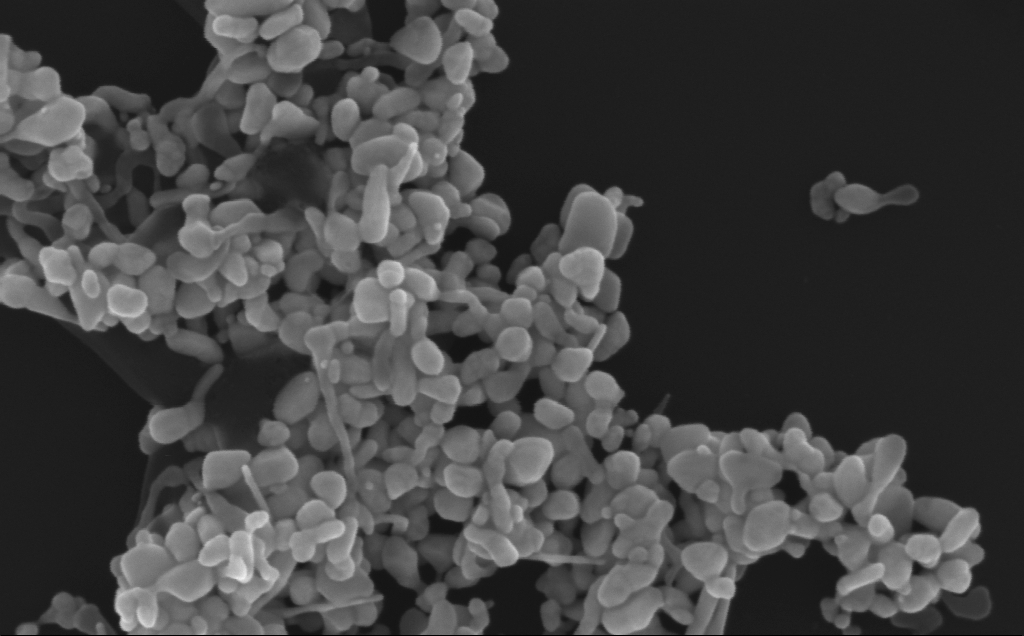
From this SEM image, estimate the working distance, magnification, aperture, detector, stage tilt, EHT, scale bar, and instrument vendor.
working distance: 3 mm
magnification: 219.14 K X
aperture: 30 µm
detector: InLens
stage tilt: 0°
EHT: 10 kV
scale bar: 200 nm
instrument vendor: Zeiss